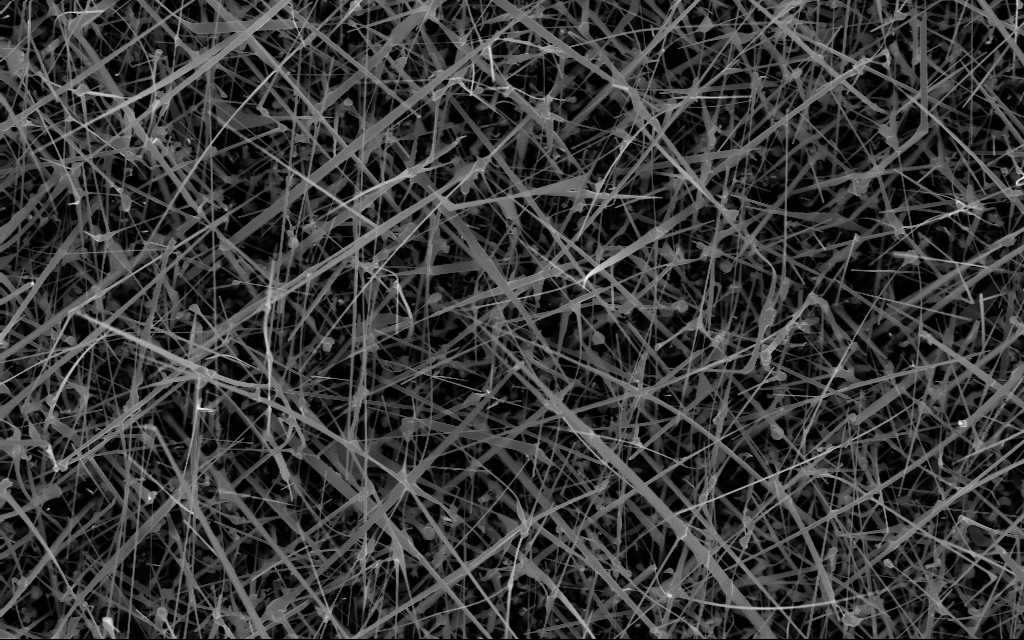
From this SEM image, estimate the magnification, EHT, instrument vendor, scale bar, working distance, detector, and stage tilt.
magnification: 20 K X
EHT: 10 kV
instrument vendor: Zeiss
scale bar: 2000 nm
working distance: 7 mm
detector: InLens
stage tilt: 0°